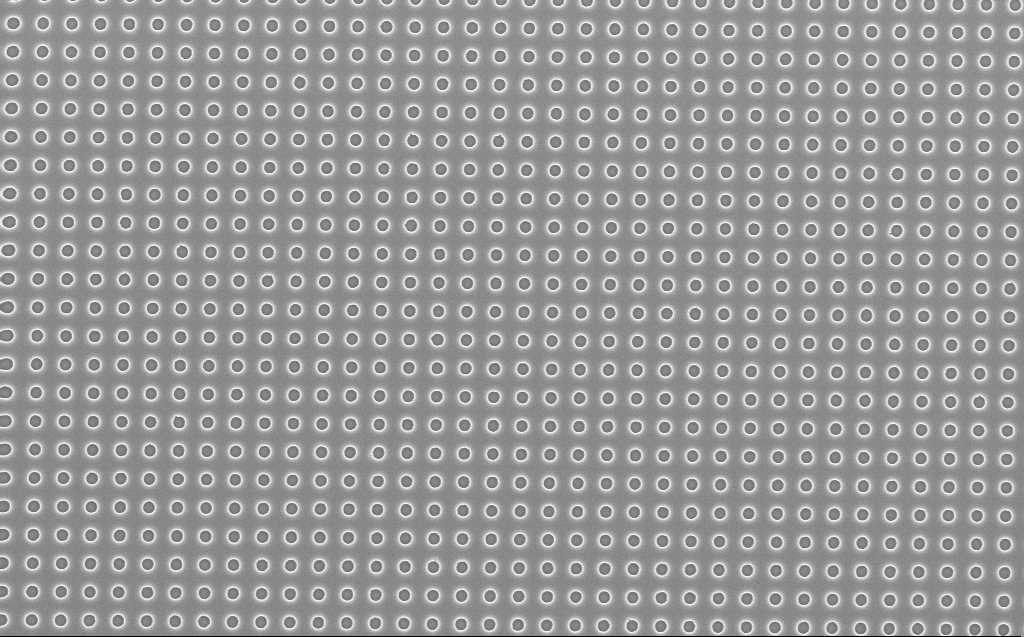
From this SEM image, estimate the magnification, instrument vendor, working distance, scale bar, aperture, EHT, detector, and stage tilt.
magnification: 10 K X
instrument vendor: Zeiss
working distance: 7 mm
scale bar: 2000 nm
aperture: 30 µm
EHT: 5 kV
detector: InLens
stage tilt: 0°